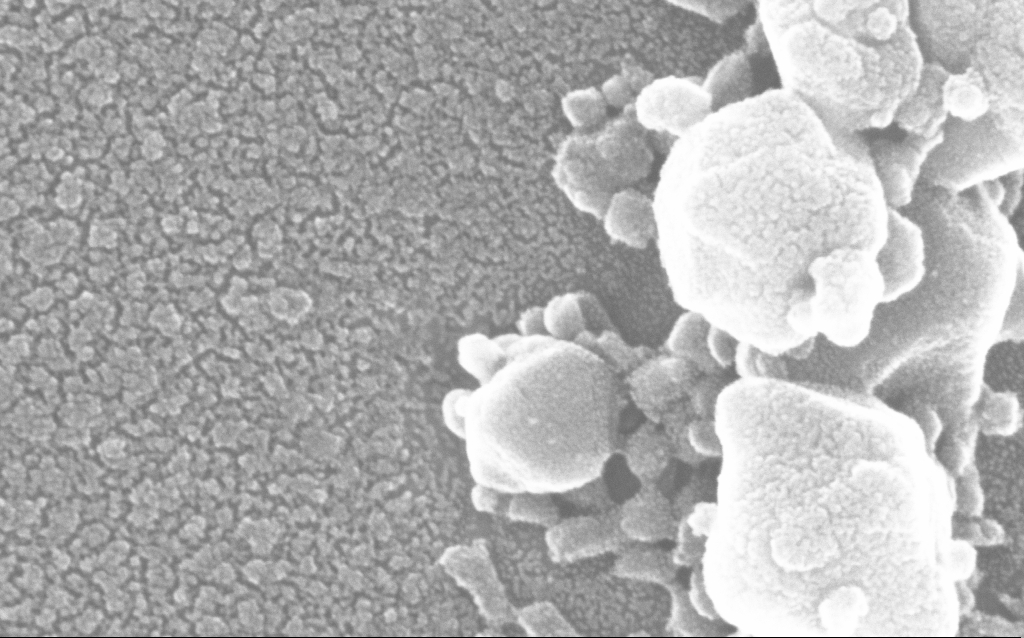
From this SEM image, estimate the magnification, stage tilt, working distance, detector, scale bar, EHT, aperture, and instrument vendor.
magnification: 500 K X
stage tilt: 0°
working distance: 1.9 mm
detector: InLens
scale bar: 100 nm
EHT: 20 kV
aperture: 30 µm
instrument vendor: Zeiss